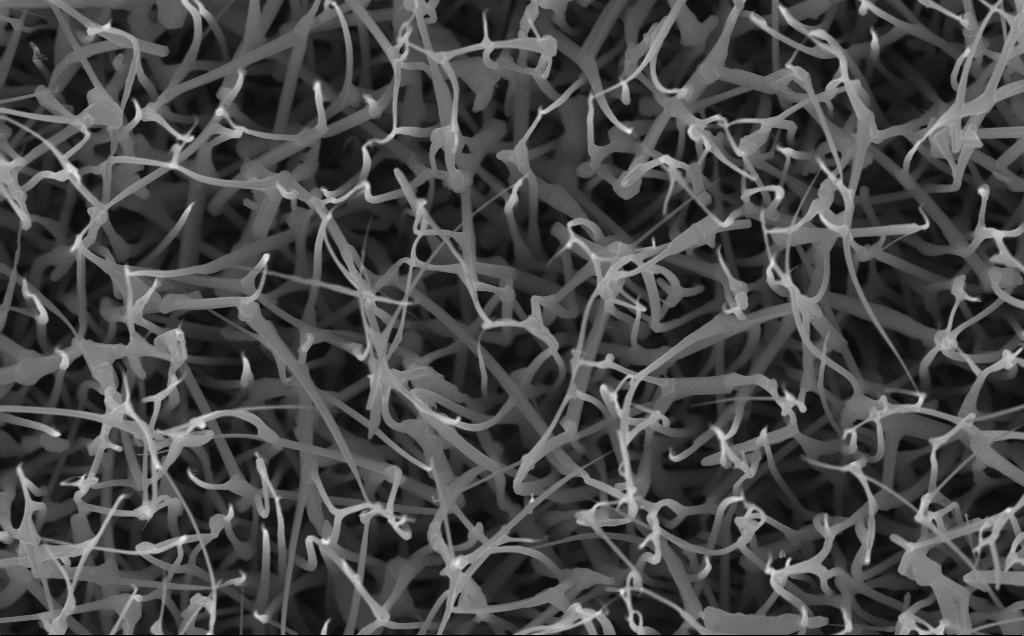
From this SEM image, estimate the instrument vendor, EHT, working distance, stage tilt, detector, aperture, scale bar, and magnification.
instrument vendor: Zeiss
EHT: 10 kV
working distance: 5 mm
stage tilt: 0°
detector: InLens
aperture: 30 µm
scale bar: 1000 nm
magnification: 40 K X